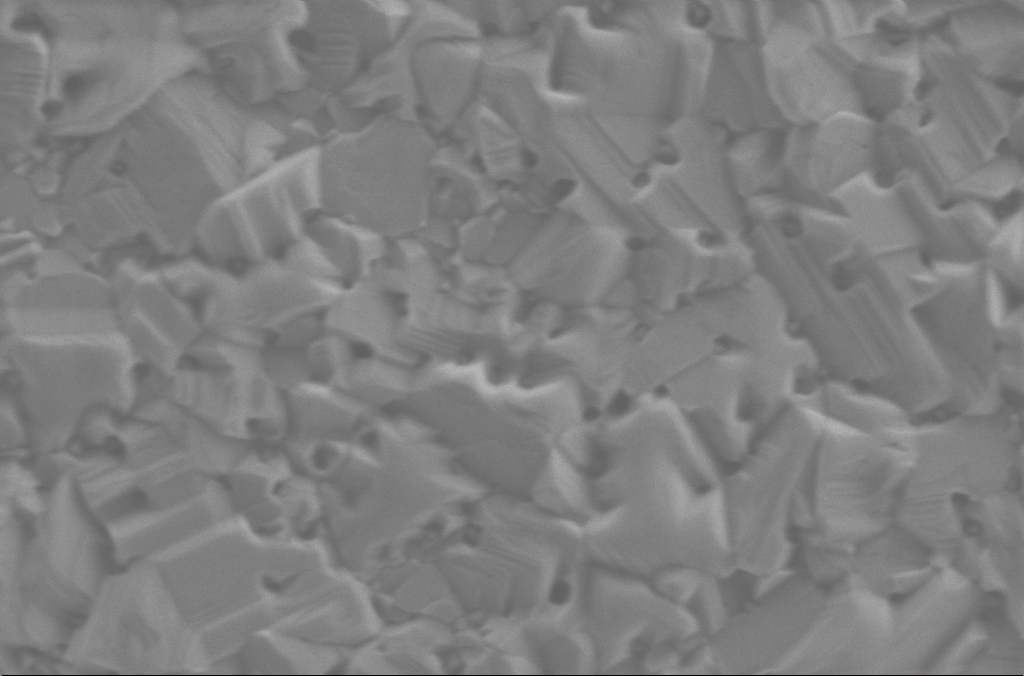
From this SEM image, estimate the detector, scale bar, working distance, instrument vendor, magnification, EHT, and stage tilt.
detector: SE2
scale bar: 200 nm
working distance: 3 mm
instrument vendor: Zeiss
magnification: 70 K X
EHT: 2 kV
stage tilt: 0°